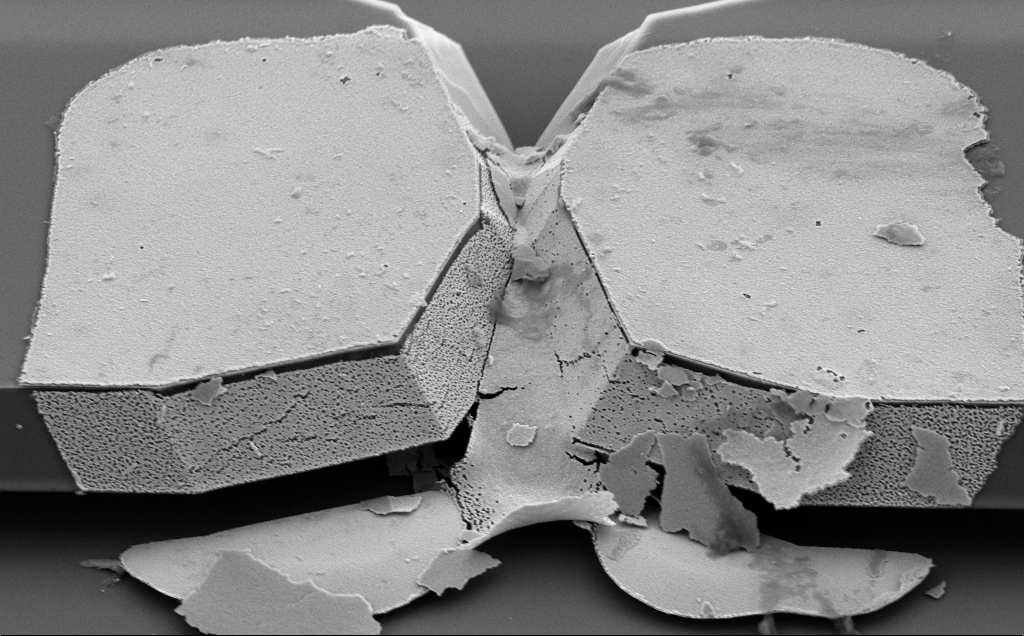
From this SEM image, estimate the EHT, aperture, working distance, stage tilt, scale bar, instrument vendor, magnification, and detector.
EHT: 5 kV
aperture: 30 µm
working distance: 10 mm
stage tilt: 50°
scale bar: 2000 nm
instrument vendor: Zeiss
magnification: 13.54 K X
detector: SE2